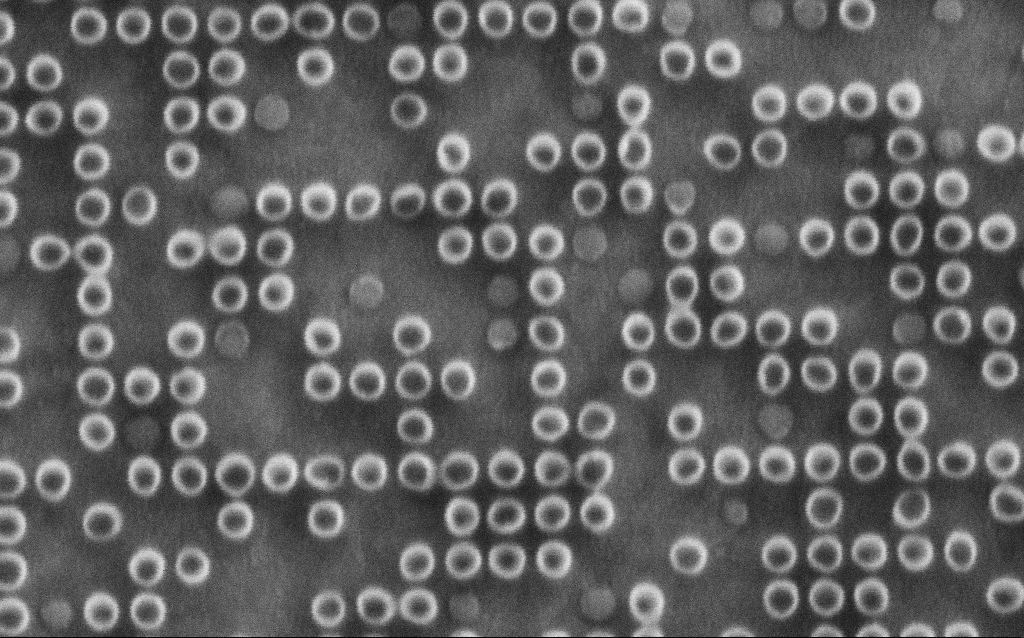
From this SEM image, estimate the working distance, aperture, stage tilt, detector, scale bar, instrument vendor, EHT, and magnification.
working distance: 5.8 mm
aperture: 30 µm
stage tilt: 0°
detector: SE2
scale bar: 200 nm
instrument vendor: Zeiss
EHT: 1.5 kV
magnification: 164.73 K X